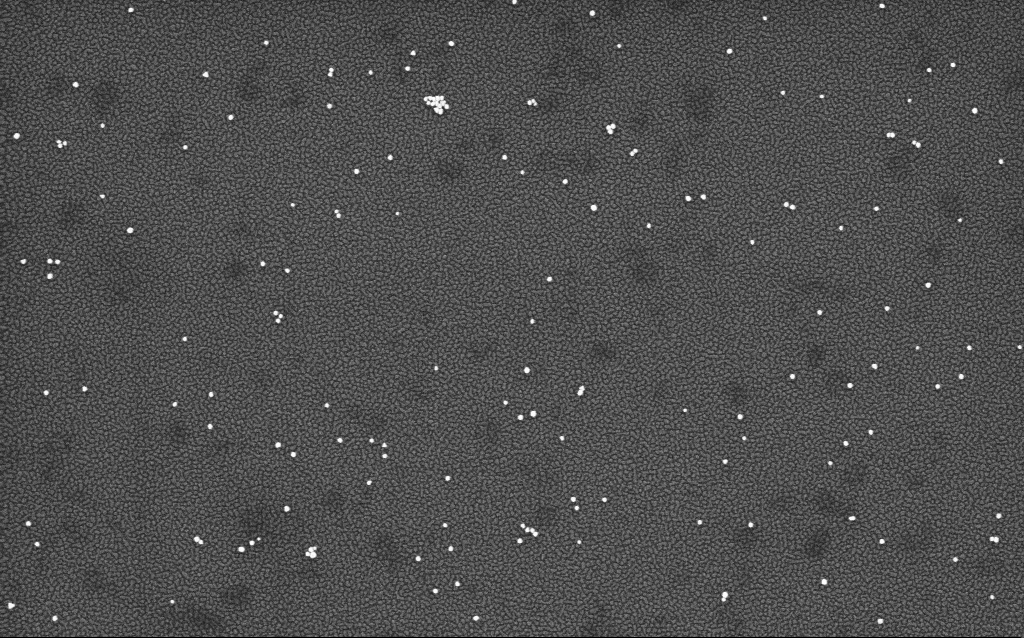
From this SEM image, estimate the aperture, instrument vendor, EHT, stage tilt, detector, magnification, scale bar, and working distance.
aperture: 30 µm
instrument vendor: Zeiss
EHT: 4 kV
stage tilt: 0°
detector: InLens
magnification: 100 K X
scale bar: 200 nm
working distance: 1.7 mm